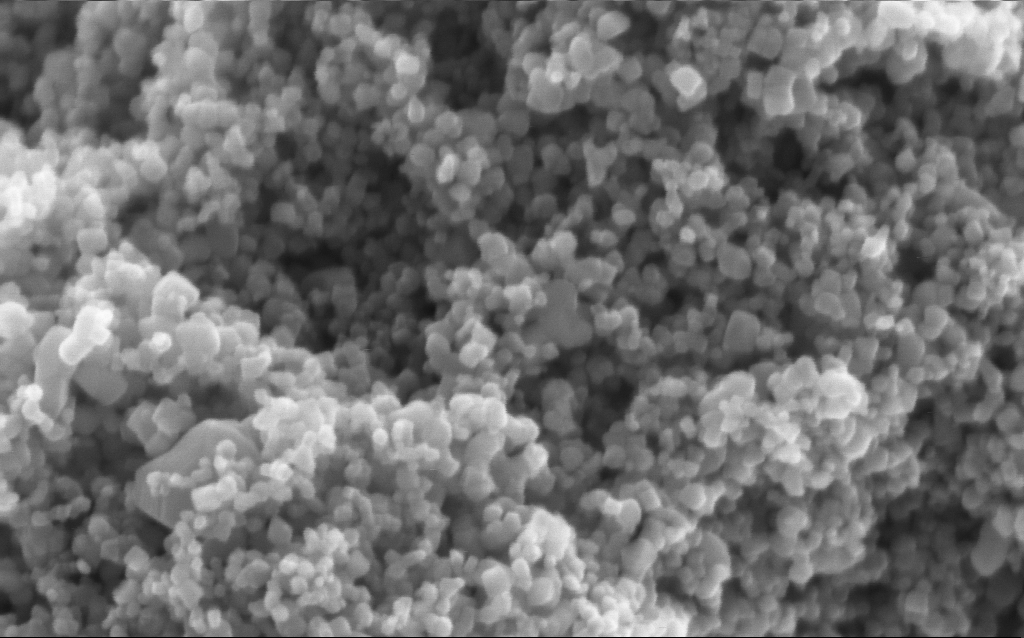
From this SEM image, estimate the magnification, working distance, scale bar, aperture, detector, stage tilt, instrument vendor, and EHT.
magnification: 282.06 K X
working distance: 4 mm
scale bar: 200 nm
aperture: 30 µm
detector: InLens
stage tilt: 0°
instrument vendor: Zeiss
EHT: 5 kV